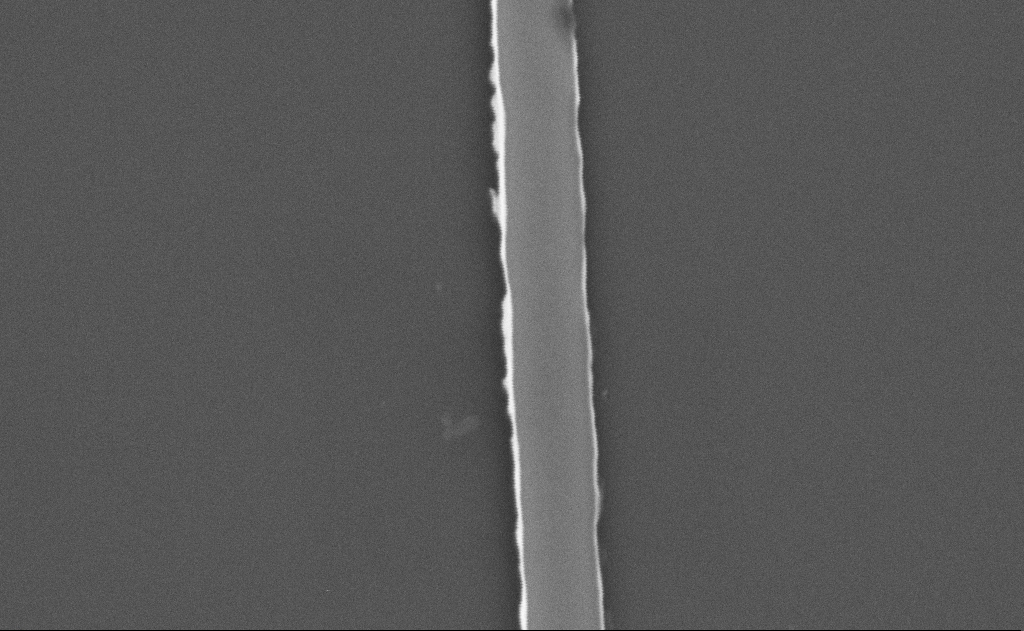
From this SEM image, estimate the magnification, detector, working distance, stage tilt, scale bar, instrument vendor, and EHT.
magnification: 62.06 K X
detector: SE2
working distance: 15 mm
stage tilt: -0°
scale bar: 1000 nm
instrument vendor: Zeiss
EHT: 5 kV